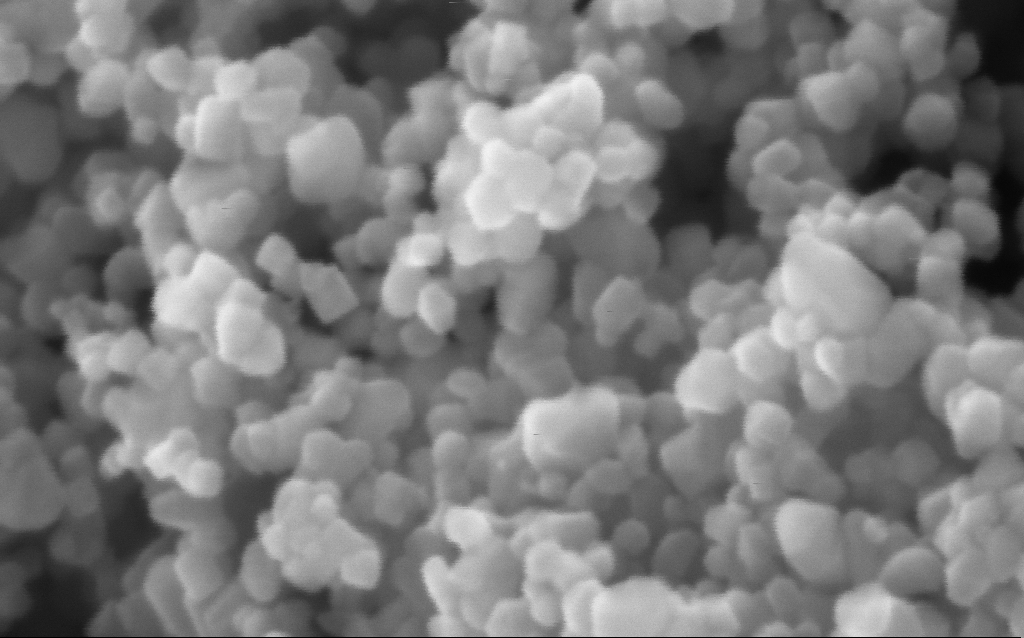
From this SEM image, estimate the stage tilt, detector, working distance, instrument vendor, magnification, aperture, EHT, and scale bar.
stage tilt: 0°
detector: InLens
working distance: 3.4 mm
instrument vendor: Zeiss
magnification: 716 K X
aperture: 30 µm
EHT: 5 kV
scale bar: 100 nm